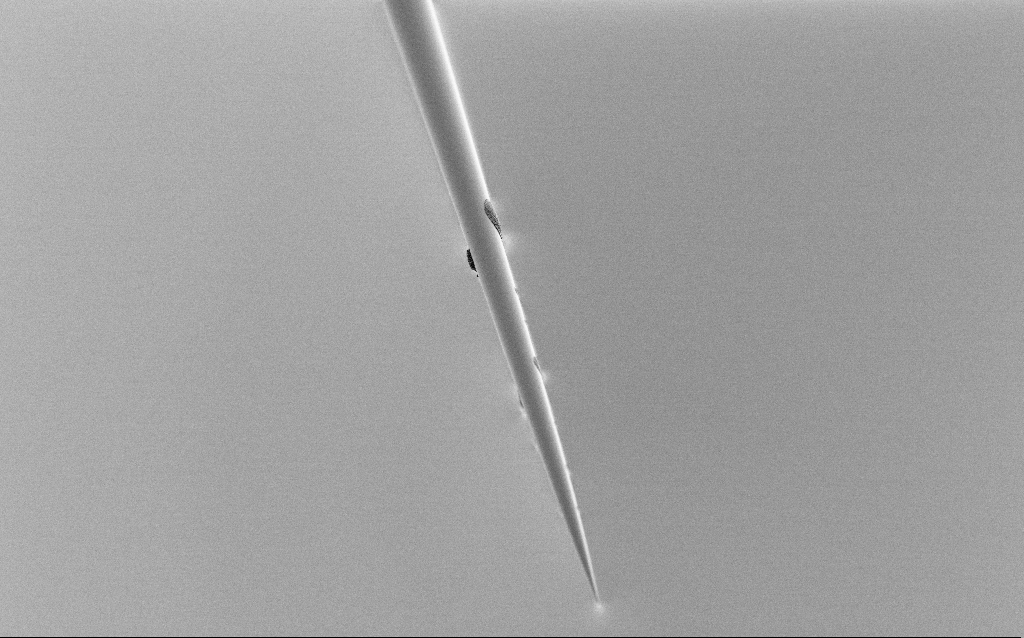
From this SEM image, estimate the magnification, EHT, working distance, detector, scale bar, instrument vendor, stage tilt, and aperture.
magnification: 0.5 K X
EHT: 1 kV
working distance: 6 mm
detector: SE2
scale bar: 100000 nm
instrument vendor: Zeiss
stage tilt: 45°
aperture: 30 µm